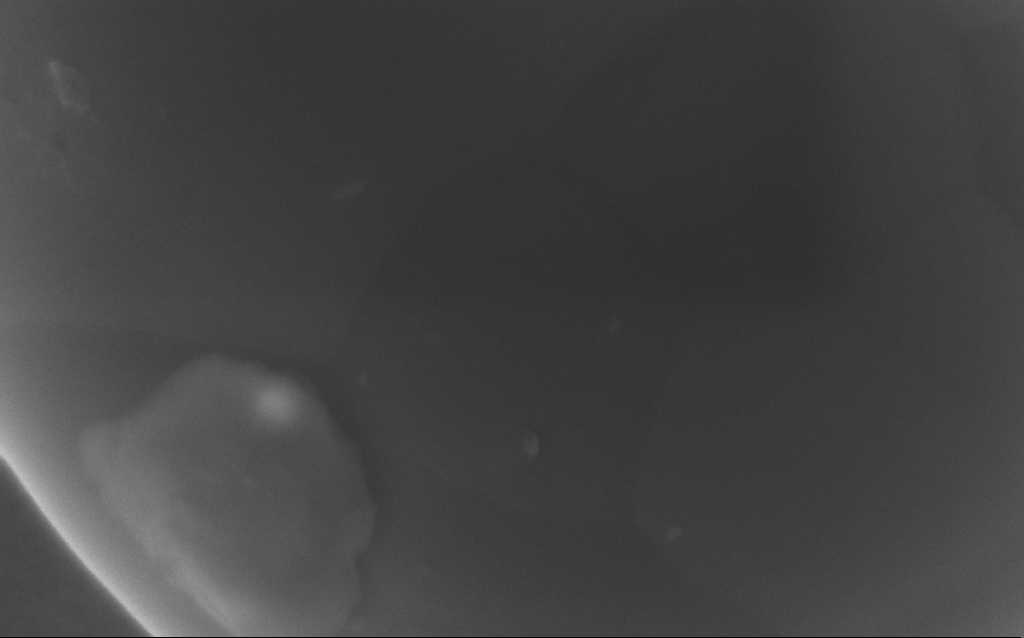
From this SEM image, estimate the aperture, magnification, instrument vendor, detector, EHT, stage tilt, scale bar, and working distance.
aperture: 30 µm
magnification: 300.65 K X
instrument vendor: Zeiss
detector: InLens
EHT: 5 kV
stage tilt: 0°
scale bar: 200 nm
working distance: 3 mm